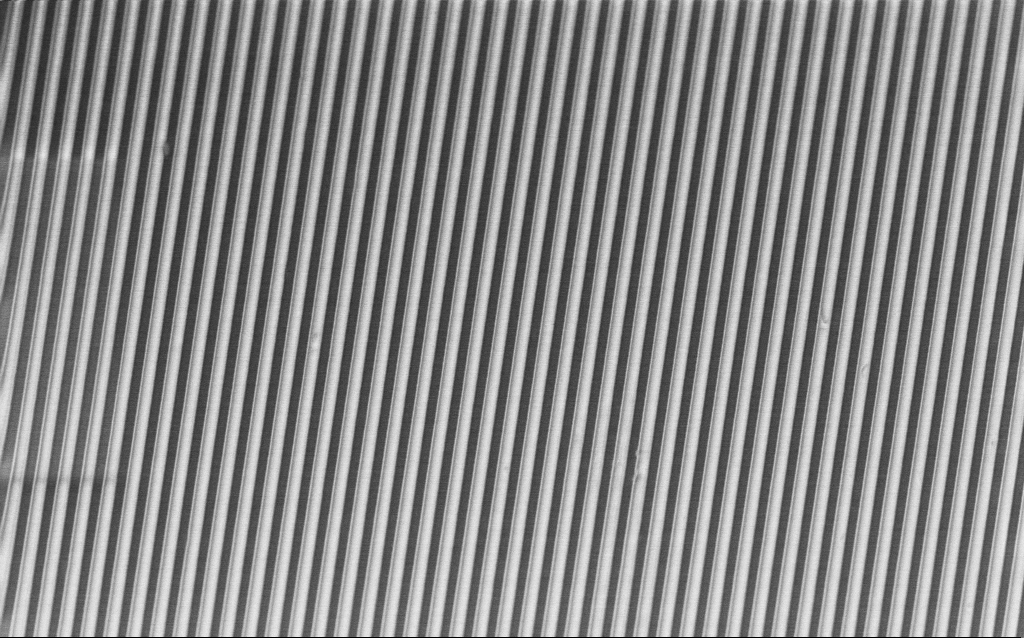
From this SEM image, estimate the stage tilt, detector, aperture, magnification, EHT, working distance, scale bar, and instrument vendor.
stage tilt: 45°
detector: InLens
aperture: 30 µm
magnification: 16.92 K X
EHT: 2 kV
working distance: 4.1 mm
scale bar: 2000 nm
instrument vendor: Zeiss